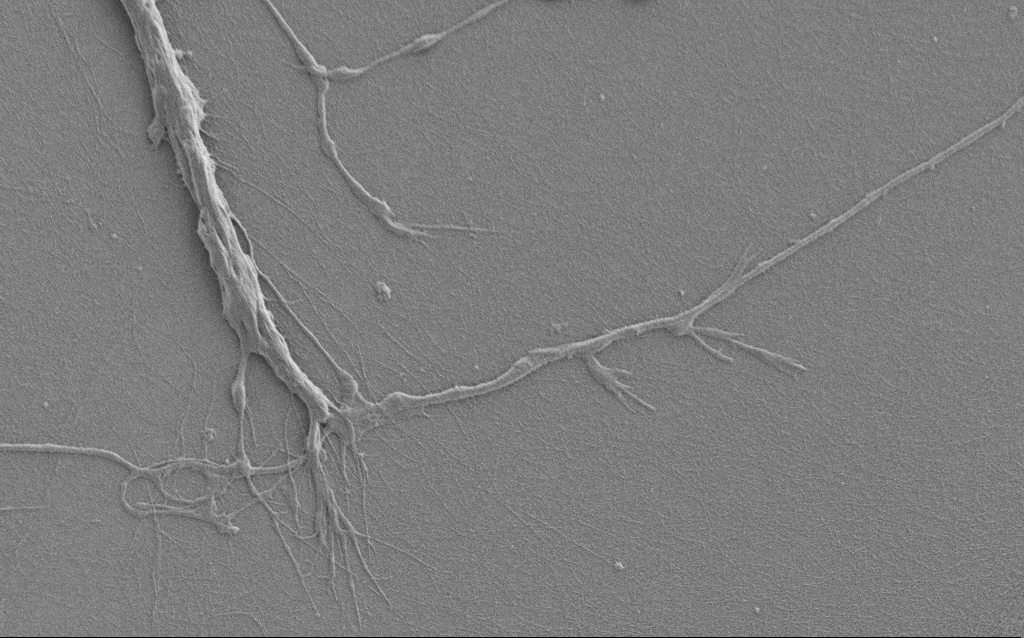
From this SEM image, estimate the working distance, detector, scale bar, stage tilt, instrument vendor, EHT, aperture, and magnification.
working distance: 6 mm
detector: SE2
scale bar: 10000 nm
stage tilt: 0°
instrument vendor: Zeiss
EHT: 1 kV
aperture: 30 µm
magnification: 5 K X